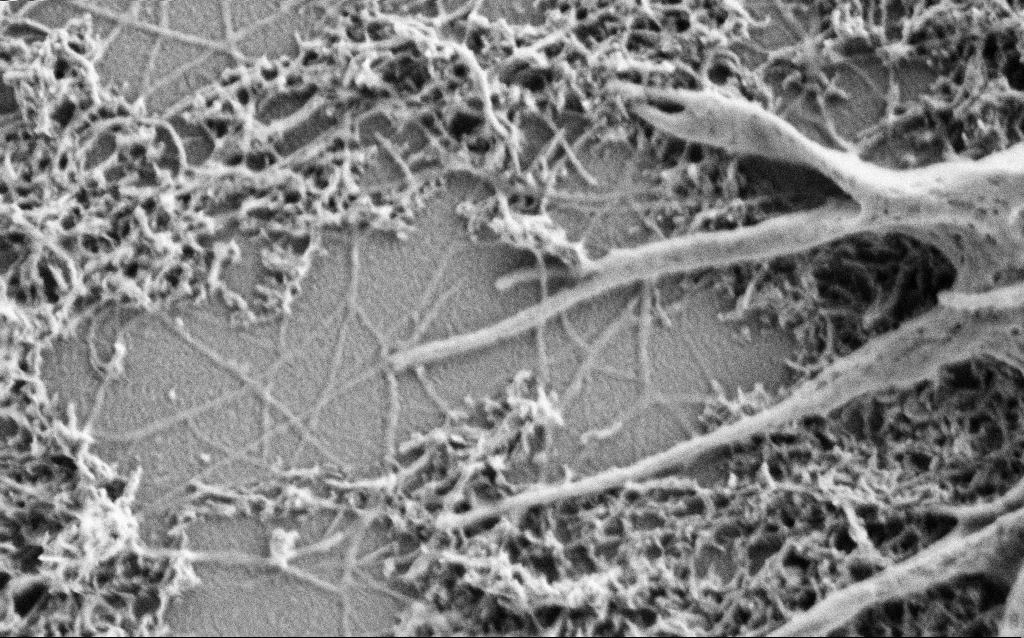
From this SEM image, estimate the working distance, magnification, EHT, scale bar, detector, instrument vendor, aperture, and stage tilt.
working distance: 4 mm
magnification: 50 K X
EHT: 1 kV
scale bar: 1000 nm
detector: SE2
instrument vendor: Zeiss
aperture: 30 µm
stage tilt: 0°